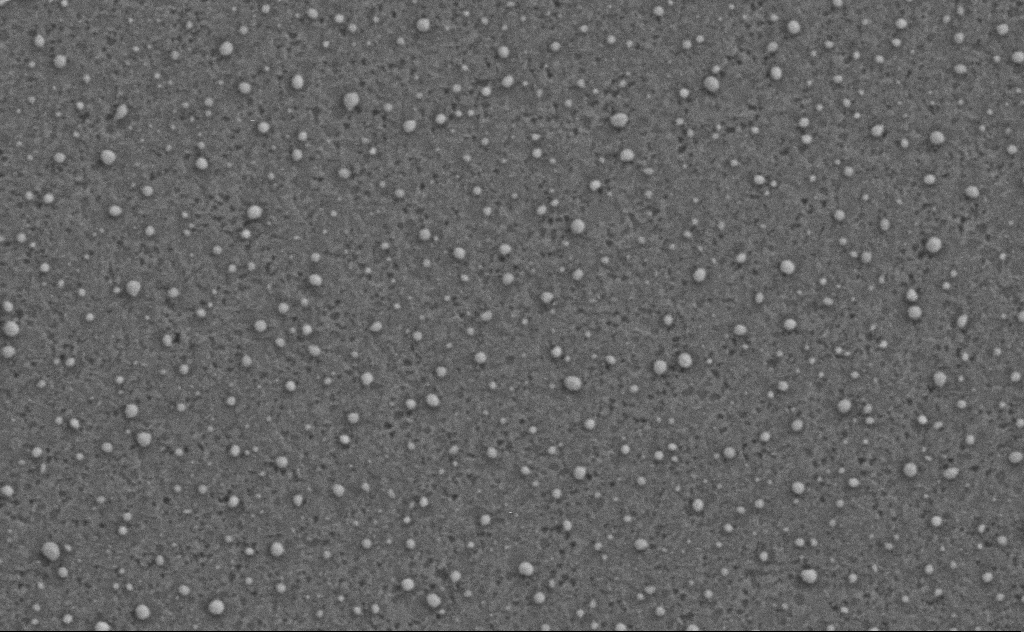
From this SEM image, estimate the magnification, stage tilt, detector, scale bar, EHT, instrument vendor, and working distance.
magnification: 80 K X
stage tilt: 0°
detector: SE2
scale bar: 200 nm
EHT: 3 kV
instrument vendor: Zeiss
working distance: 4 mm